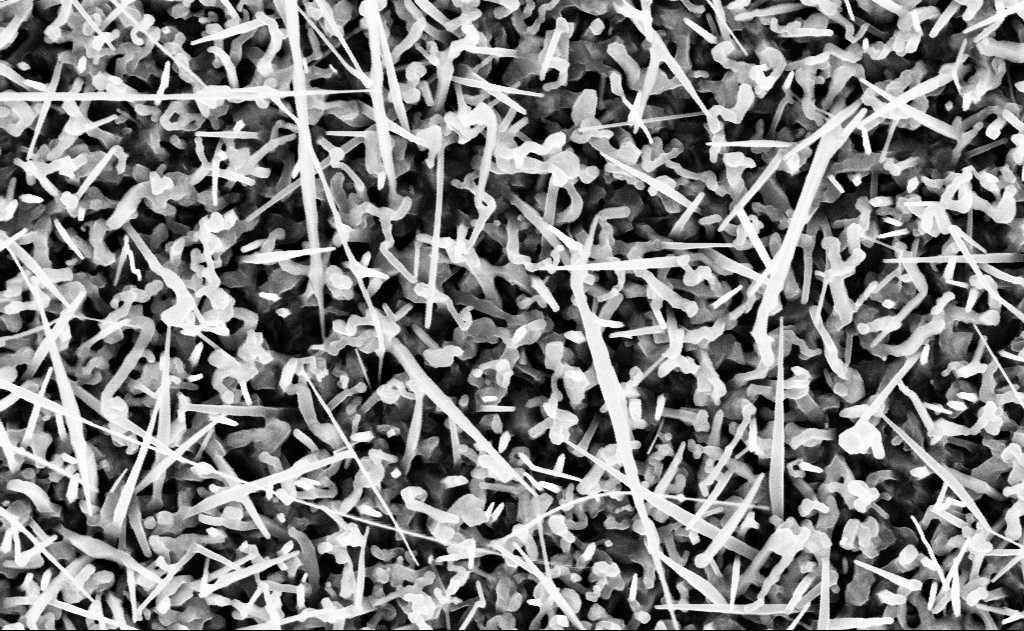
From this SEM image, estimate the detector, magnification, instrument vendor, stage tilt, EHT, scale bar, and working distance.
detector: InLens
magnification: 40 K X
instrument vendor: Zeiss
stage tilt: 0°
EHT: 10 kV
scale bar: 1000 nm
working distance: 15 mm